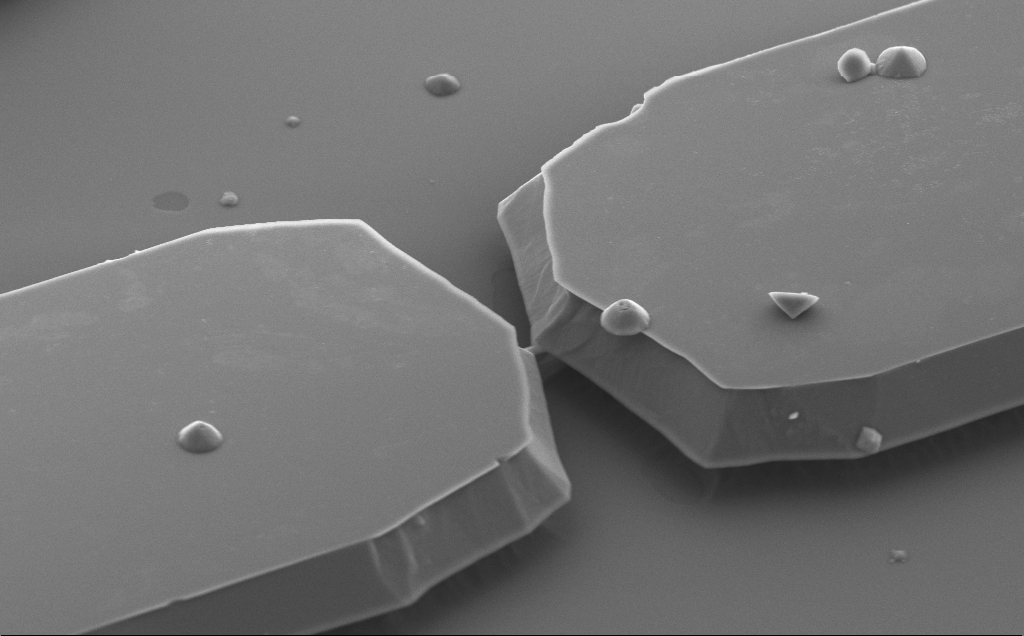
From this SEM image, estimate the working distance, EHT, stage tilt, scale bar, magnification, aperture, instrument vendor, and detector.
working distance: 10 mm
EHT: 5 kV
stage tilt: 50°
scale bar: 2000 nm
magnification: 9.54 K X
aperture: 30 µm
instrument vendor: Zeiss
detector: SE2